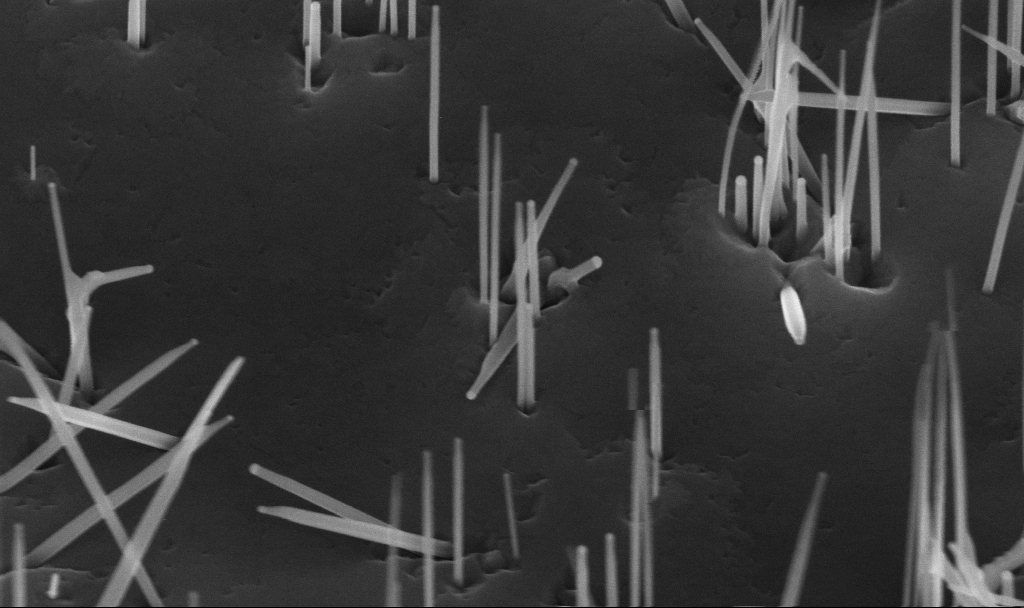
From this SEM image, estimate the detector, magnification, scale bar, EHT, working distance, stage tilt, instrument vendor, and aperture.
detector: InLens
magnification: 104.25 K X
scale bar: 200 nm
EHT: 10 kV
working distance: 5.6 mm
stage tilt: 45°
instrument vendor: Zeiss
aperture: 30 µm